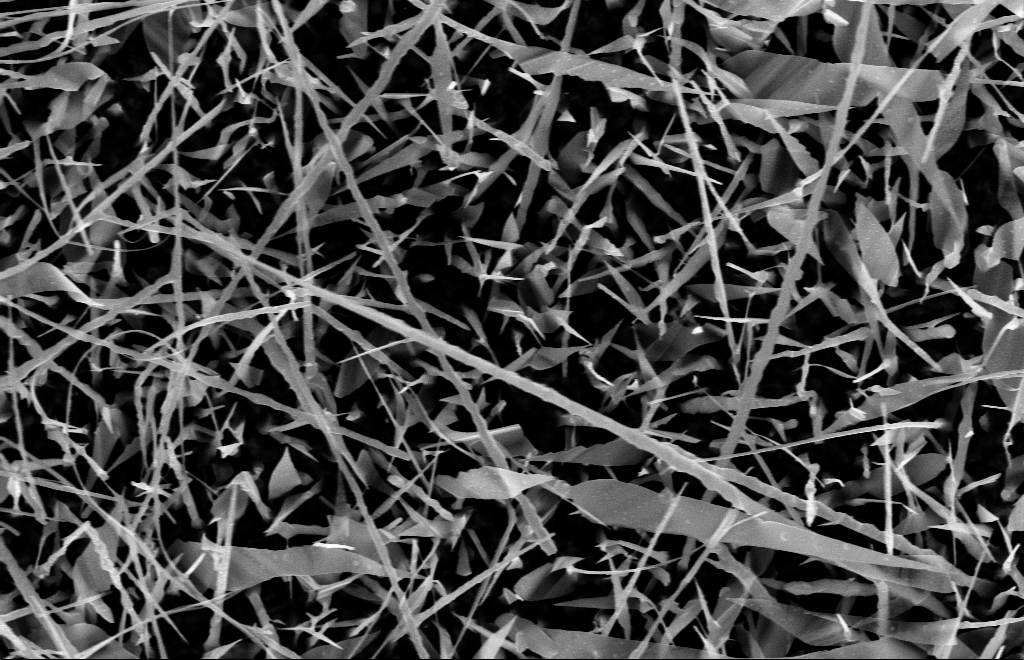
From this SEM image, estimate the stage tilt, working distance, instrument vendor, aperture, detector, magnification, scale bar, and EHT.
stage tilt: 0°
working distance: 15 mm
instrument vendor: Zeiss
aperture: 30 µm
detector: InLens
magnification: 20 K X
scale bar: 2000 nm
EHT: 10 kV